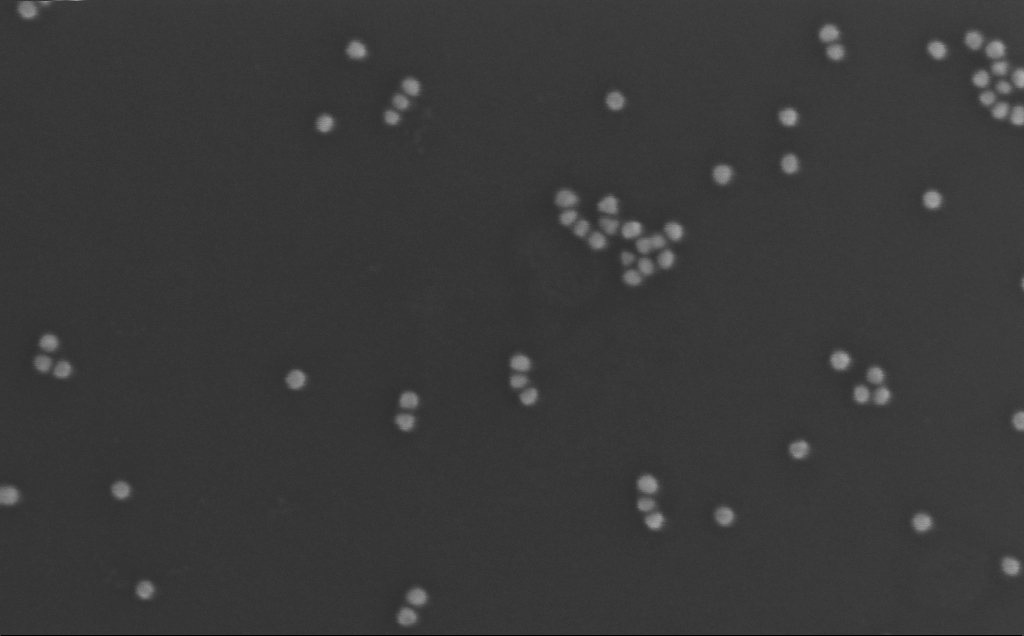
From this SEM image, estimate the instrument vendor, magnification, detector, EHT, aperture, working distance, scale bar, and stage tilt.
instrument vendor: Zeiss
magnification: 406.34 K X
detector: InLens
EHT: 10 kV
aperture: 30 µm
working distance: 3 mm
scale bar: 100 nm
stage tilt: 0°